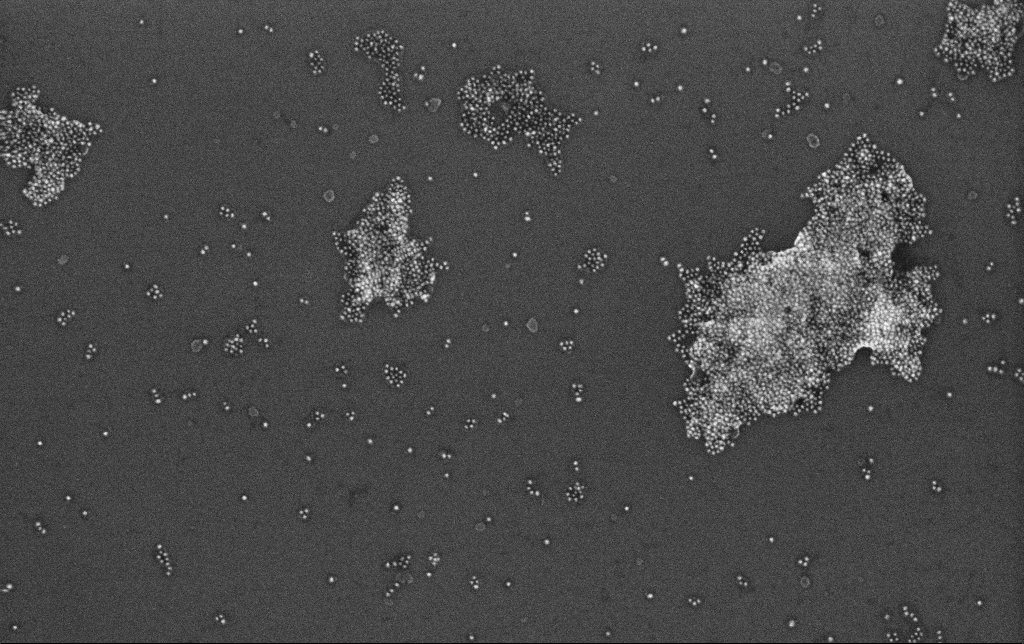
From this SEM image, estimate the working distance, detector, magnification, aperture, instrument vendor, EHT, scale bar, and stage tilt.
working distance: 3.4 mm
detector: InLens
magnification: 100 K X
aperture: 30 µm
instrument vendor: Zeiss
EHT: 10 kV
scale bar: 200 nm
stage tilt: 0°